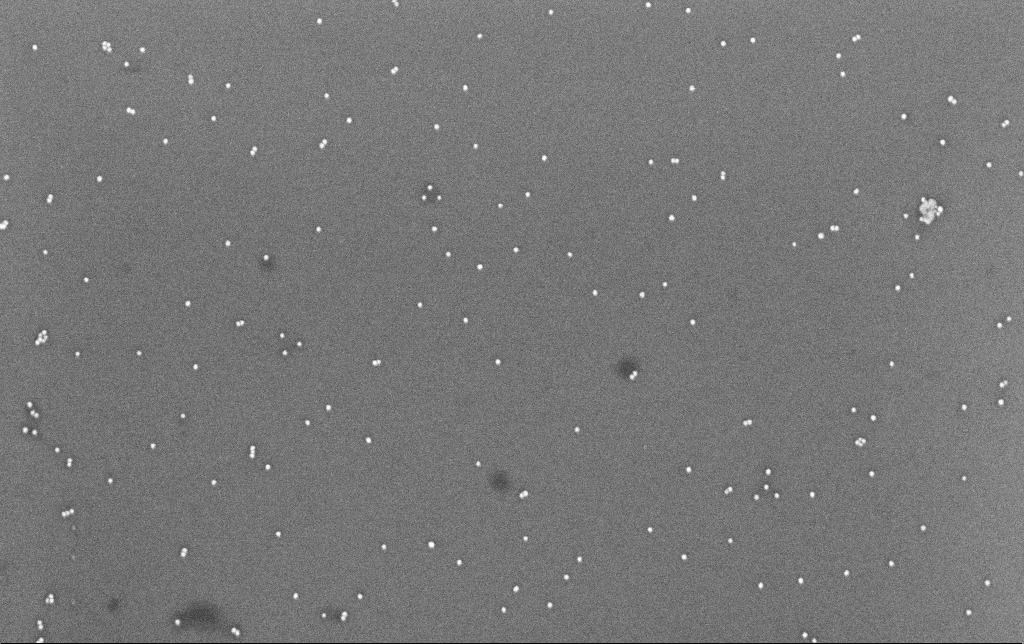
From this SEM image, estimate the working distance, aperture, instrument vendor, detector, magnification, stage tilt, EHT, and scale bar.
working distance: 6.6 mm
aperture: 30 µm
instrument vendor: Zeiss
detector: InLens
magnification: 100 K X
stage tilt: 0°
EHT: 8 kV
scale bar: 200 nm